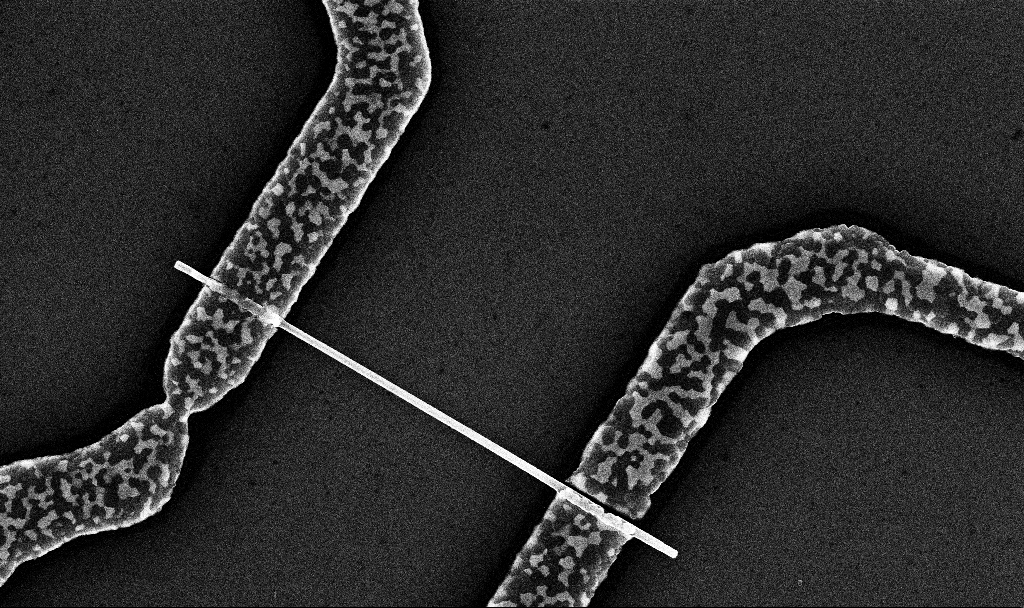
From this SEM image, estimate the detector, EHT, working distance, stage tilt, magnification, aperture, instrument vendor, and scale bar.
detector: InLens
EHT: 10 kV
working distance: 6.7 mm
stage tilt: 0°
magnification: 35.51 K X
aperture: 30 µm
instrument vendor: Zeiss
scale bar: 1000 nm